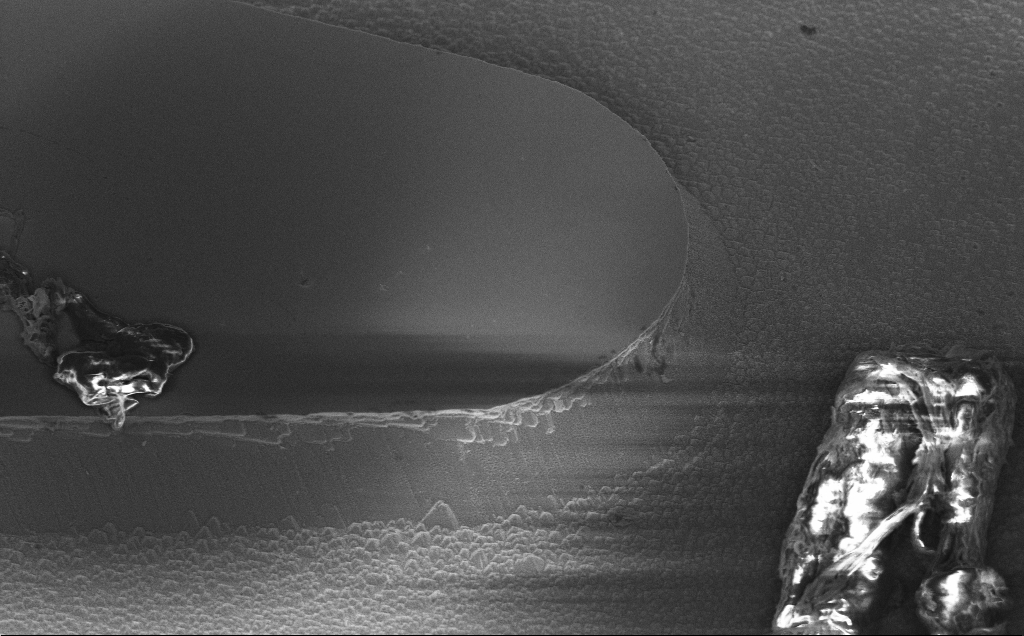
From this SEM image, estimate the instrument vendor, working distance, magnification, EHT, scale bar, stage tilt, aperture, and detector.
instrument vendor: Zeiss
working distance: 13 mm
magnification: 2.72 K X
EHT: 5 kV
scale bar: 10000 nm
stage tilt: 45°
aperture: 30 µm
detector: InLens